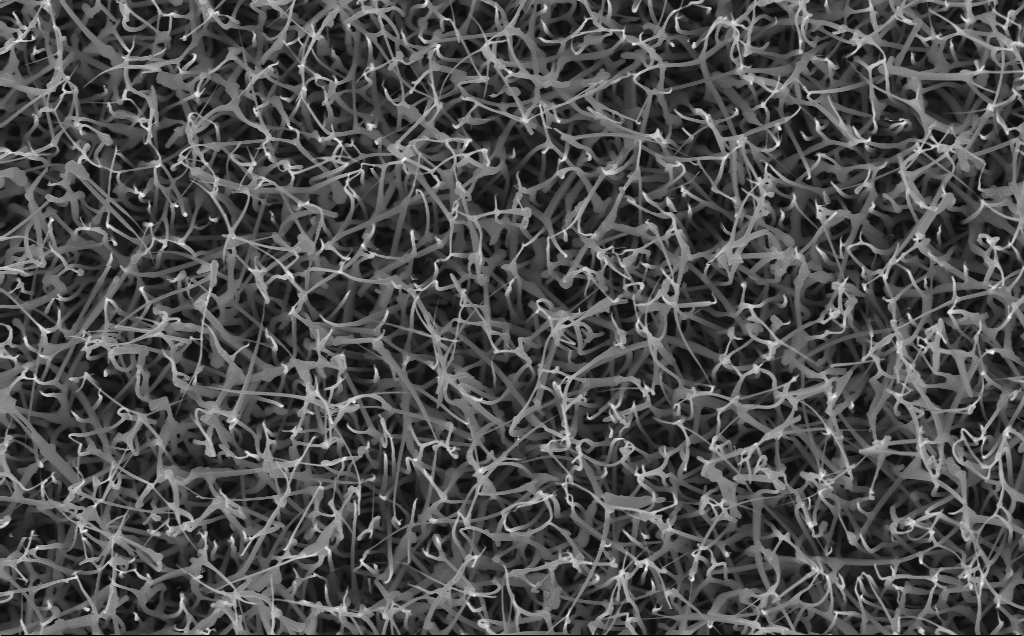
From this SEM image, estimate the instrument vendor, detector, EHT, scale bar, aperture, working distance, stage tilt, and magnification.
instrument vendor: Zeiss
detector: InLens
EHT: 10 kV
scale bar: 2000 nm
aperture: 30 µm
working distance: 5 mm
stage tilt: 0°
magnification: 20 K X